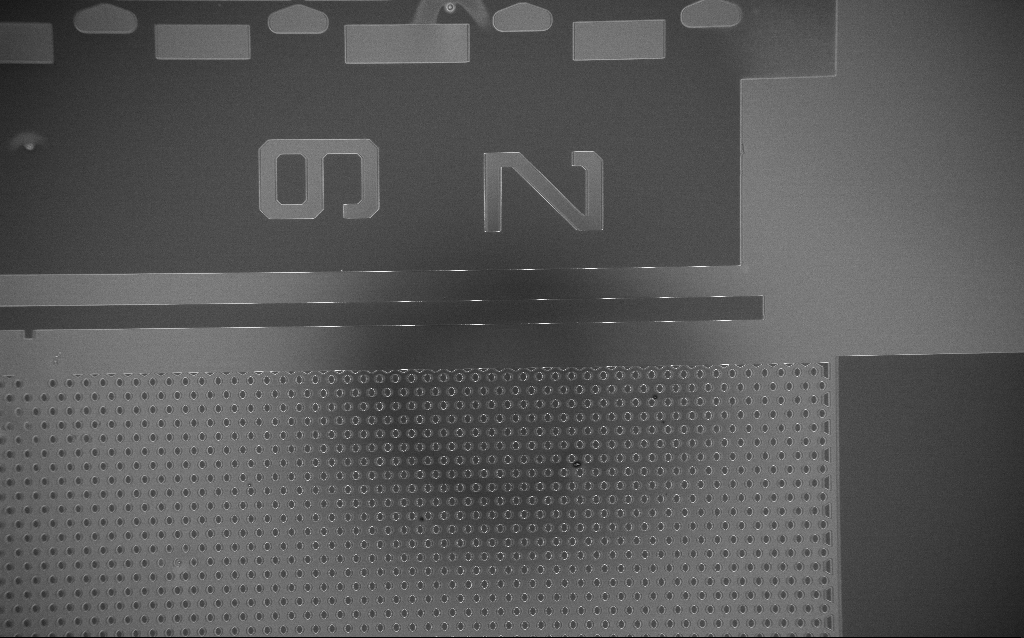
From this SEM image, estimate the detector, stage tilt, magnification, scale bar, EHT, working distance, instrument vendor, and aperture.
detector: InLens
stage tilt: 0°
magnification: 0.173 K X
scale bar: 200000 nm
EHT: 3 kV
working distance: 5 mm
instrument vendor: Zeiss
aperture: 30 µm